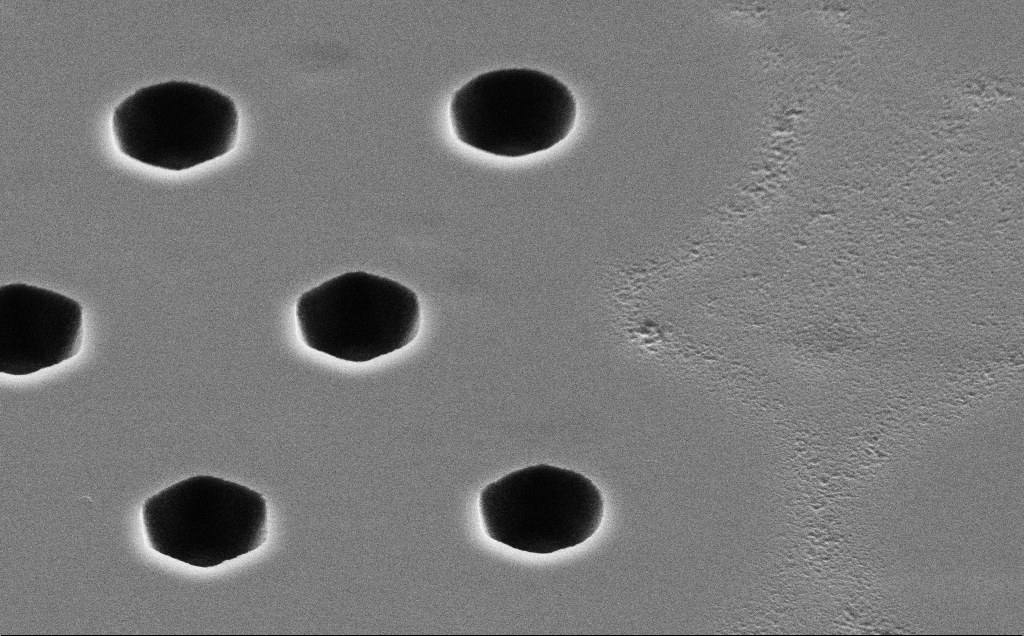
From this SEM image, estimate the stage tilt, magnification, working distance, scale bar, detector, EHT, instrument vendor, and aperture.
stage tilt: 45°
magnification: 19.48 K X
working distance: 10 mm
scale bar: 2000 nm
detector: SE2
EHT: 5 kV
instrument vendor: Zeiss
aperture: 30 µm